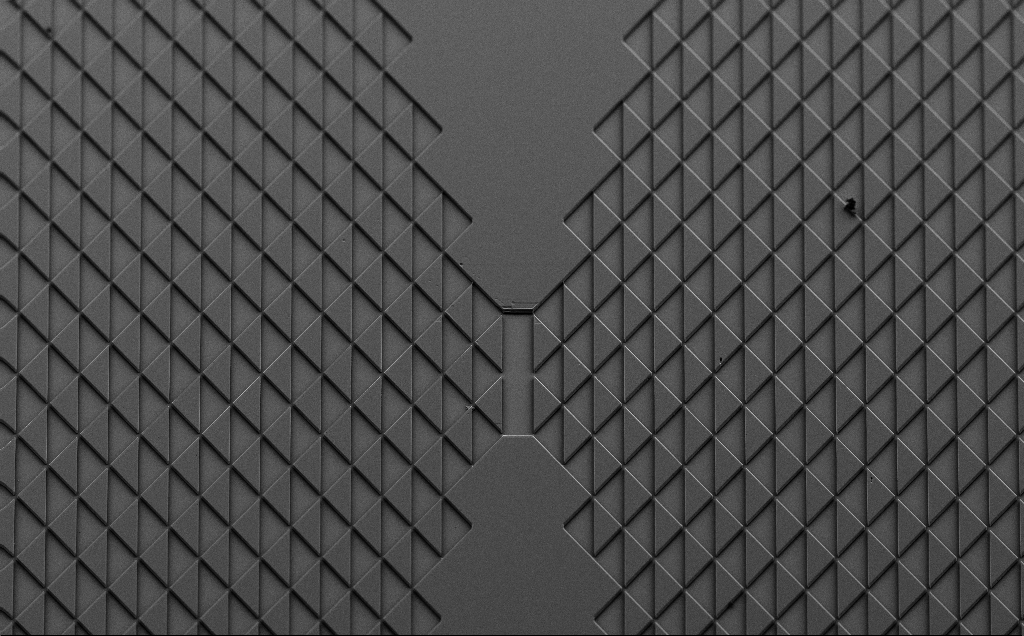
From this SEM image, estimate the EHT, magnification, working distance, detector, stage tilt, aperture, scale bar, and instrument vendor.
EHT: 5 kV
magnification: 0.316 K X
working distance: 9 mm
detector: SE2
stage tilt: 40°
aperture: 30 µm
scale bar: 100000 nm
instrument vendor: Zeiss